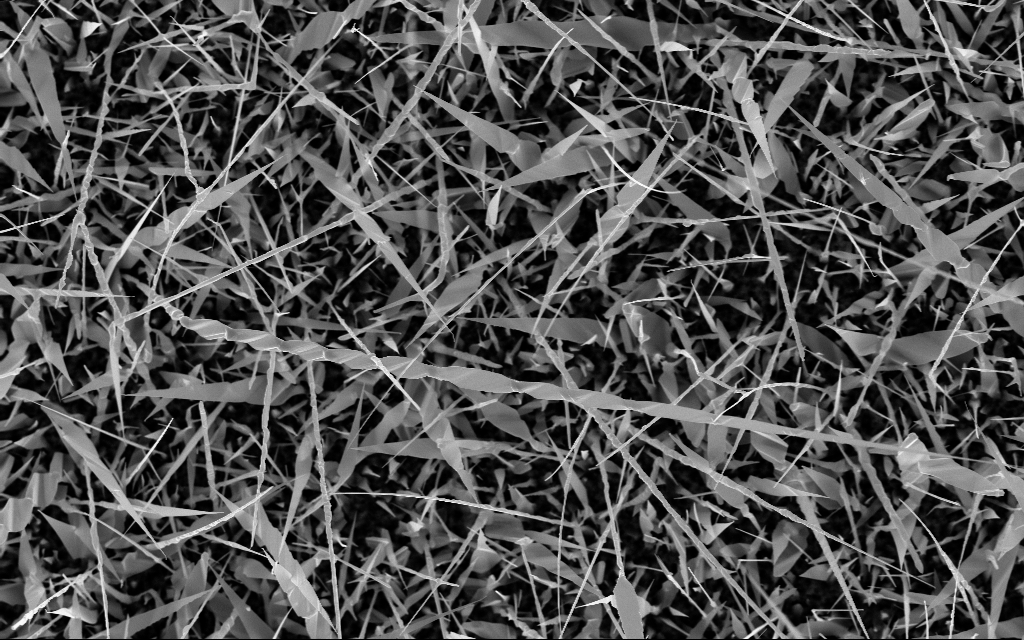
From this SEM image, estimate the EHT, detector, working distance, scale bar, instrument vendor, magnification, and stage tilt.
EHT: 10 kV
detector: InLens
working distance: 6 mm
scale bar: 2000 nm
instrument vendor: Zeiss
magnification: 10.63 K X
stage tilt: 0°